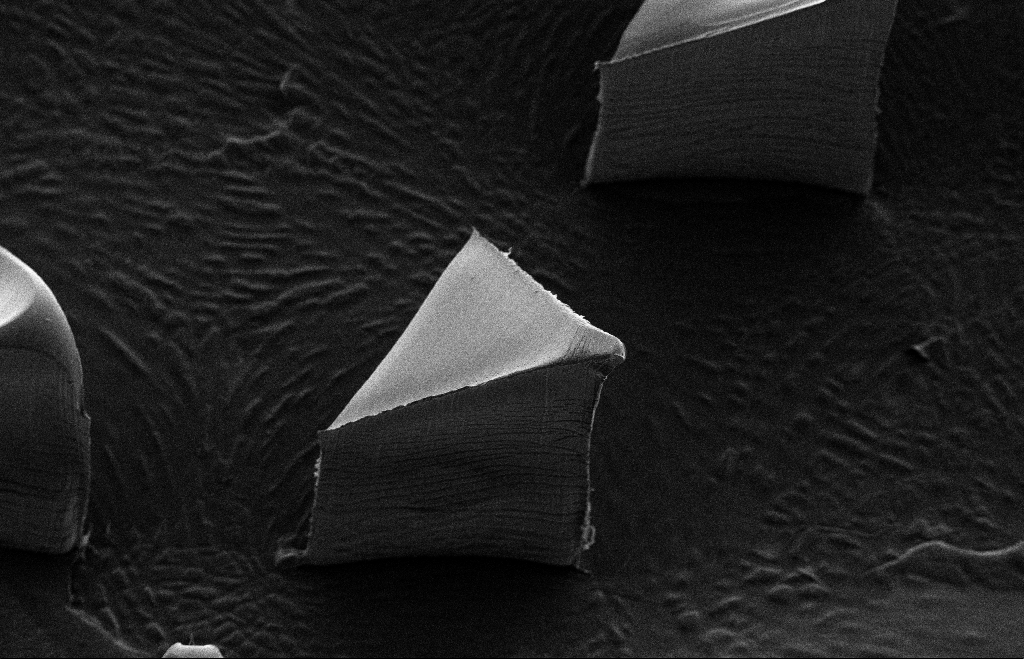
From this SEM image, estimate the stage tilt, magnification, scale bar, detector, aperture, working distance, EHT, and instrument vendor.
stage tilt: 14.7°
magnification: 0.398 K X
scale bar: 100000 nm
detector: SE2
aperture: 30 µm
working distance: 13 mm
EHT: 5 kV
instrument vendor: Zeiss